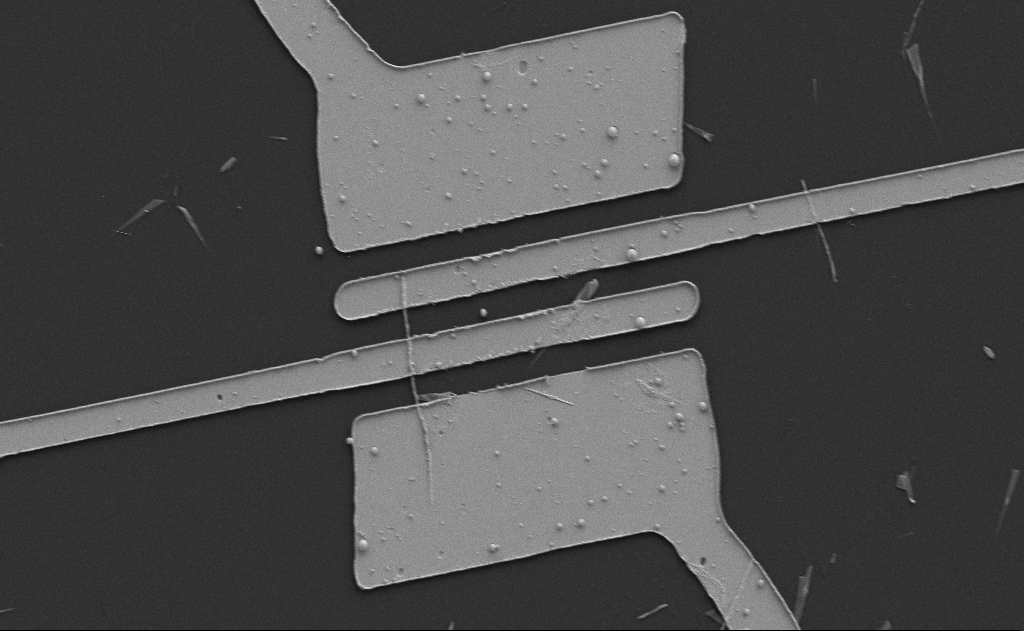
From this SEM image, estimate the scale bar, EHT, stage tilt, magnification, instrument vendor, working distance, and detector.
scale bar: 10000 nm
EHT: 5 kV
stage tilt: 0°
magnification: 4.56 K X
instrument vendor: Zeiss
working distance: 10 mm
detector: SE2